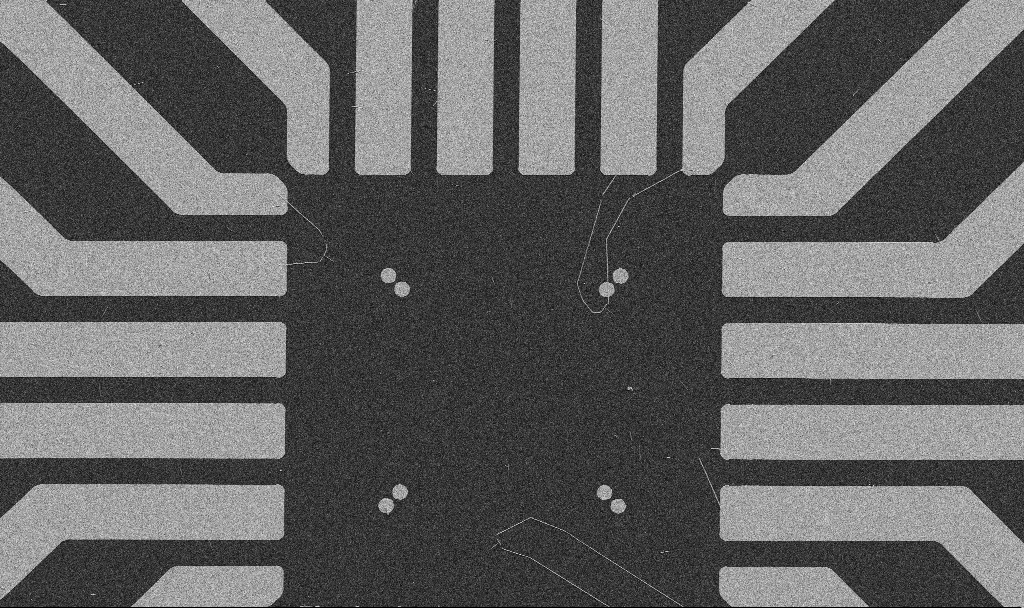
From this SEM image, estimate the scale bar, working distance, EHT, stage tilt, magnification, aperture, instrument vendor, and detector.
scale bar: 20000 nm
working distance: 10.7 mm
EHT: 5 kV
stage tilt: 0°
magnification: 1 K X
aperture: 30 µm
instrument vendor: Zeiss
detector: SE2